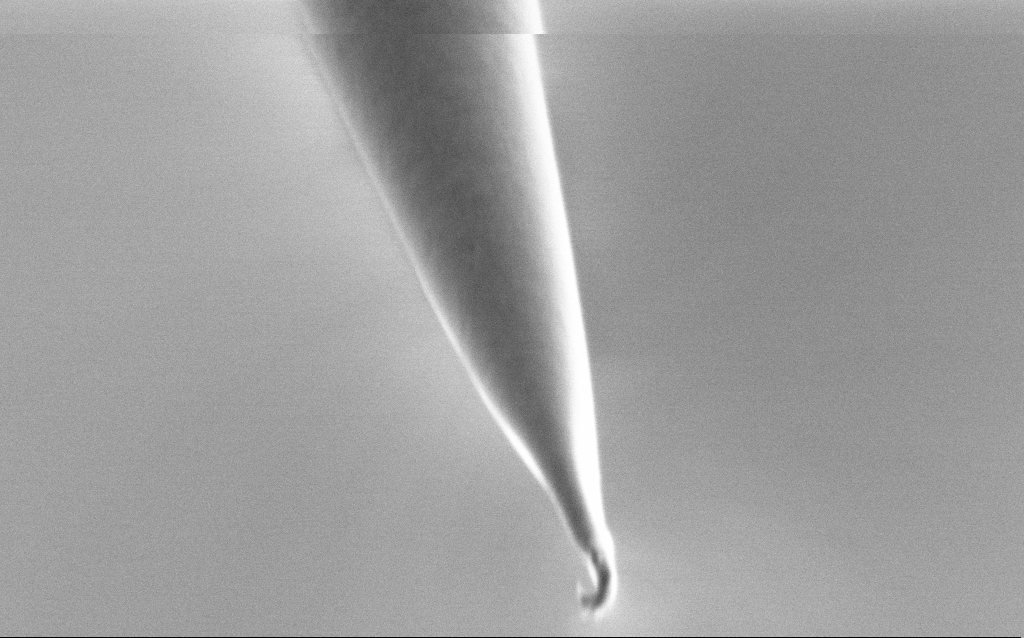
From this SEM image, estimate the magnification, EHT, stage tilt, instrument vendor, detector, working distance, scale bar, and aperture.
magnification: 100 K X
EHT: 1 kV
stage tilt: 45°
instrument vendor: Zeiss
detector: SE2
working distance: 6 mm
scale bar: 200 nm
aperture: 30 µm